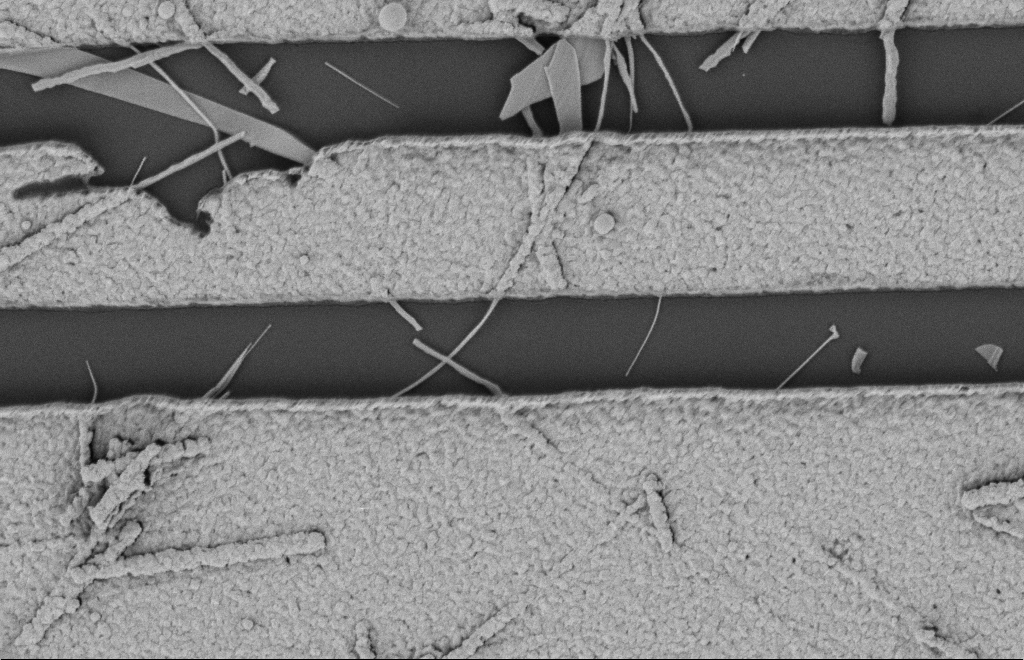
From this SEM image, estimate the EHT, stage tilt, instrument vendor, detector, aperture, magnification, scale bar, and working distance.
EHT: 2 kV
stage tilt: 0°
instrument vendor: Zeiss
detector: SE2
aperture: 20 µm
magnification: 24.07 K X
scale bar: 1000 nm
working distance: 12 mm